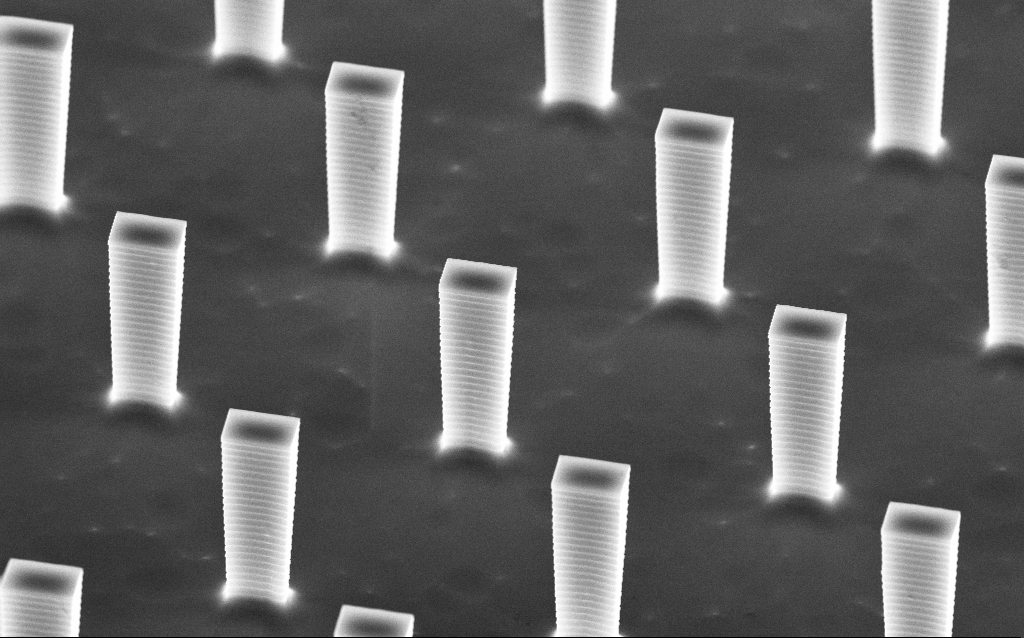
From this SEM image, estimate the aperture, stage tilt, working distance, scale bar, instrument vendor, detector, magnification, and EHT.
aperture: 30 µm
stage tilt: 45°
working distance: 5.1 mm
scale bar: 2000 nm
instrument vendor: Zeiss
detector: InLens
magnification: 10.29 K X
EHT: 10 kV